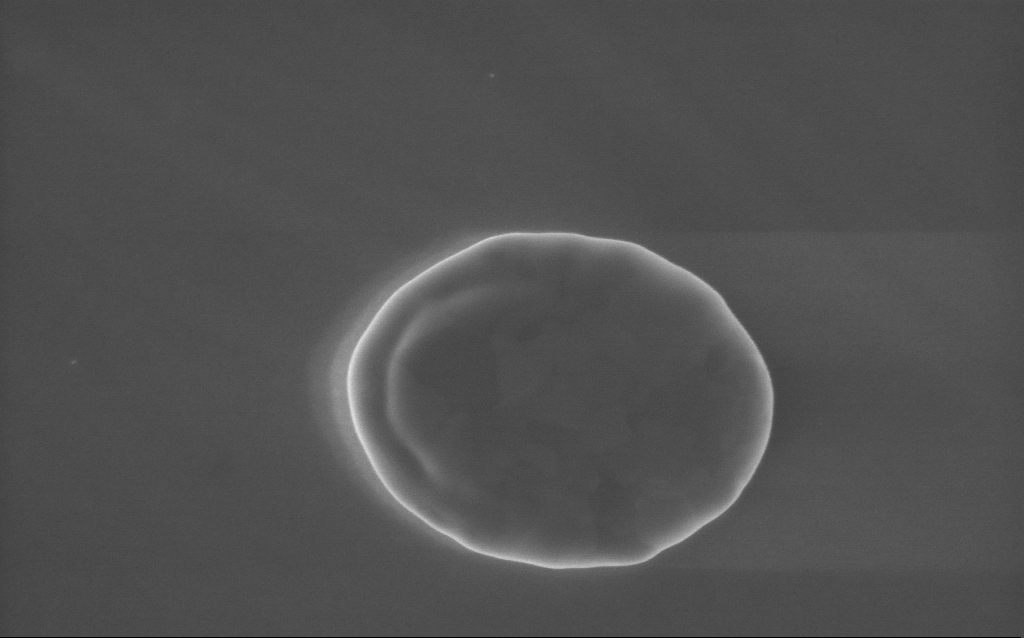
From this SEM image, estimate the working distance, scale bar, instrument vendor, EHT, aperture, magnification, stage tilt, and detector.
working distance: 3 mm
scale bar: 1000 nm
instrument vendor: Zeiss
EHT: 5 kV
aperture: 30 µm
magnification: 53 K X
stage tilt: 0°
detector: InLens